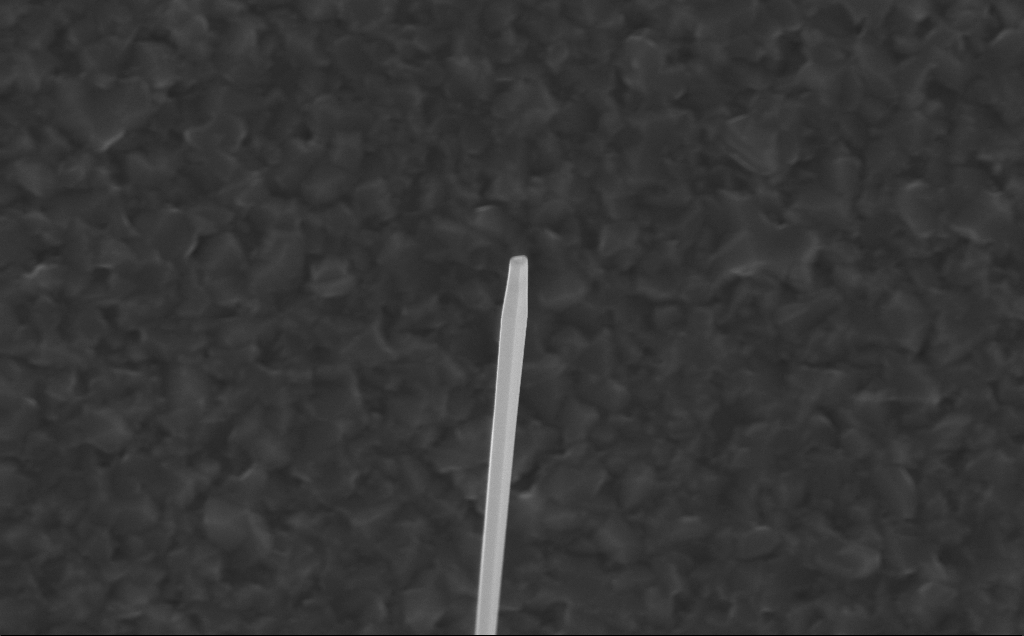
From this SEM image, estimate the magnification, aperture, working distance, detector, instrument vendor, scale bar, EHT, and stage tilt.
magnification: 40 K X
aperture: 30 µm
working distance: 5 mm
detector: InLens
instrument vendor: Zeiss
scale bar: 1000 nm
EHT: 10 kV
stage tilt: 0°